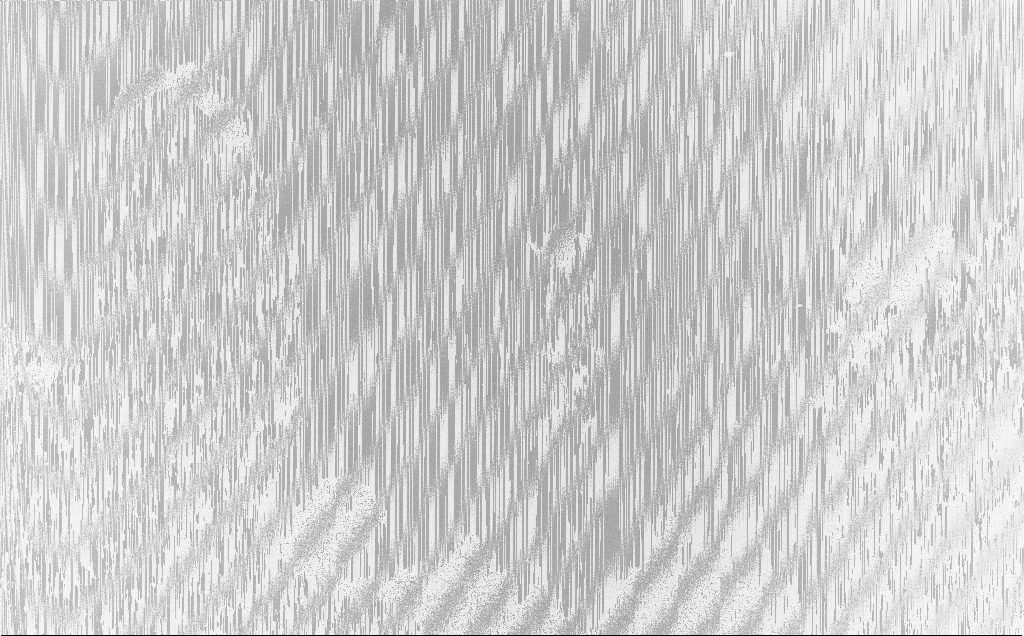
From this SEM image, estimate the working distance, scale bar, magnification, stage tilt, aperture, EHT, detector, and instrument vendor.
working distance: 6 mm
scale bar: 20000 nm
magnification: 0.913 K X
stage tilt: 0°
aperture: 30 µm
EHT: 10 kV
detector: InLens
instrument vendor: Zeiss